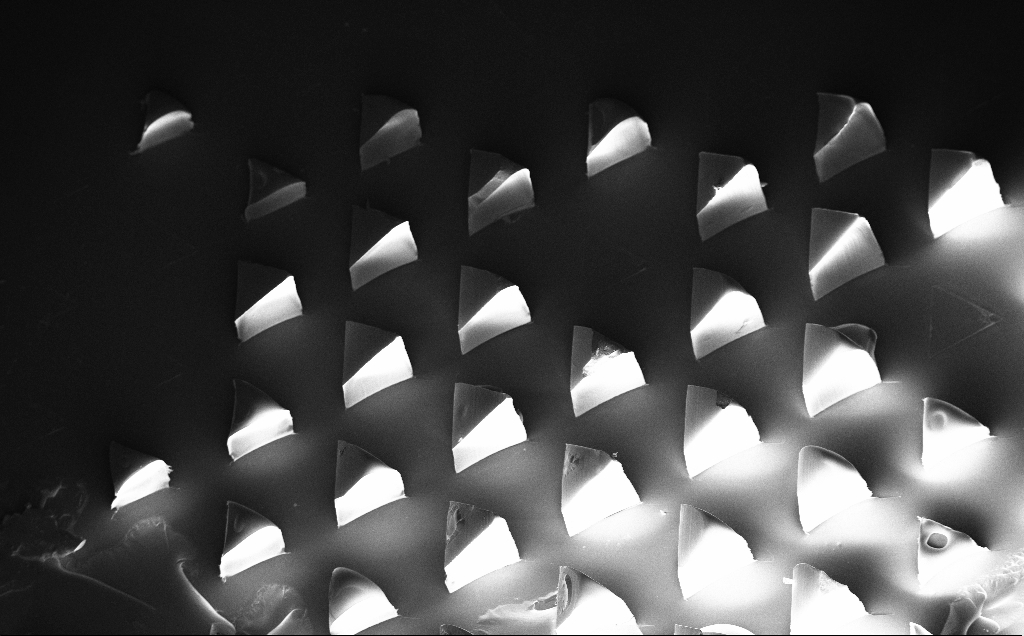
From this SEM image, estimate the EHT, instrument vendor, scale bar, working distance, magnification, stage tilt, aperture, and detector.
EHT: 10 kV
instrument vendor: Zeiss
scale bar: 200000 nm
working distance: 10 mm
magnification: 0.11 K X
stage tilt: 20°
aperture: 30 µm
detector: InLens